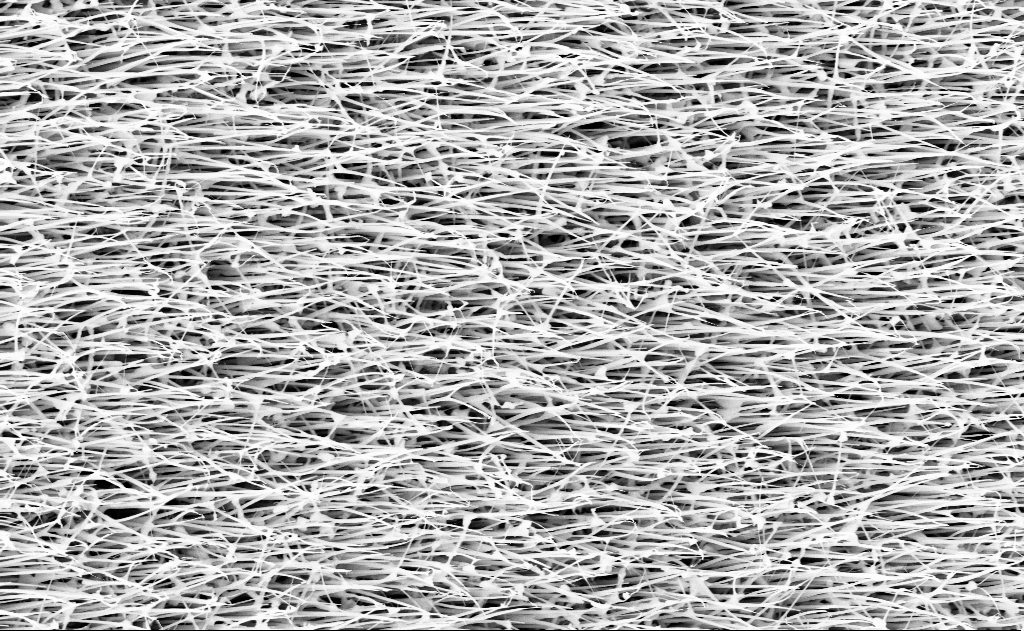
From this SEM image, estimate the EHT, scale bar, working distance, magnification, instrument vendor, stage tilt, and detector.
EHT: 10 kV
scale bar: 2000 nm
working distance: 13 mm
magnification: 20 K X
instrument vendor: Zeiss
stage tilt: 0°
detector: InLens